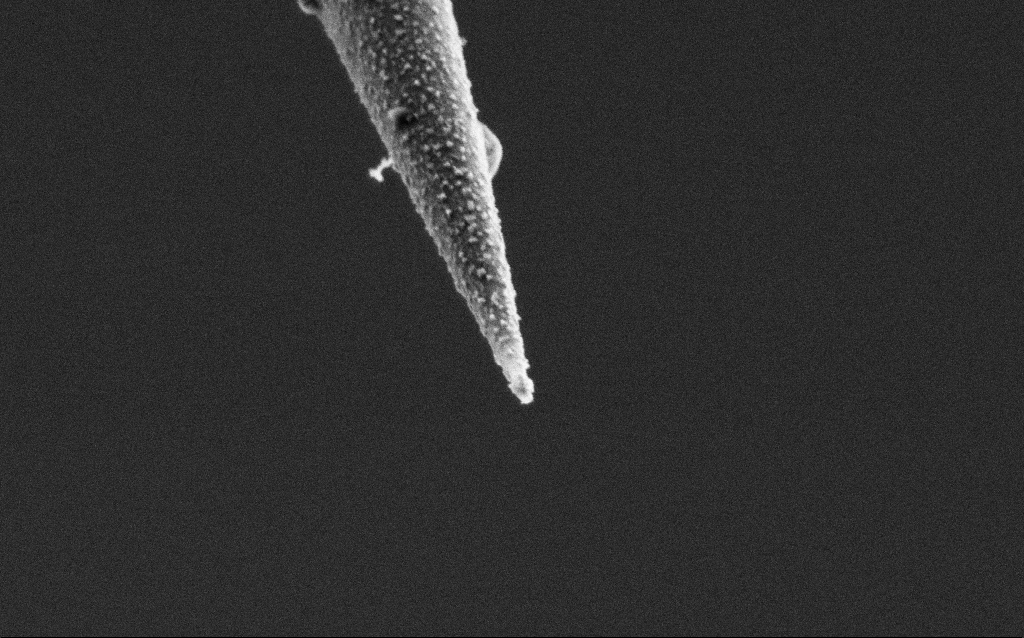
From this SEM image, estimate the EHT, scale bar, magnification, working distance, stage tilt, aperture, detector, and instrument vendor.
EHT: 2 kV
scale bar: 1000 nm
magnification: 50 K X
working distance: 7.8 mm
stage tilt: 45°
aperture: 30 µm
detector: SE2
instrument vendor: Zeiss